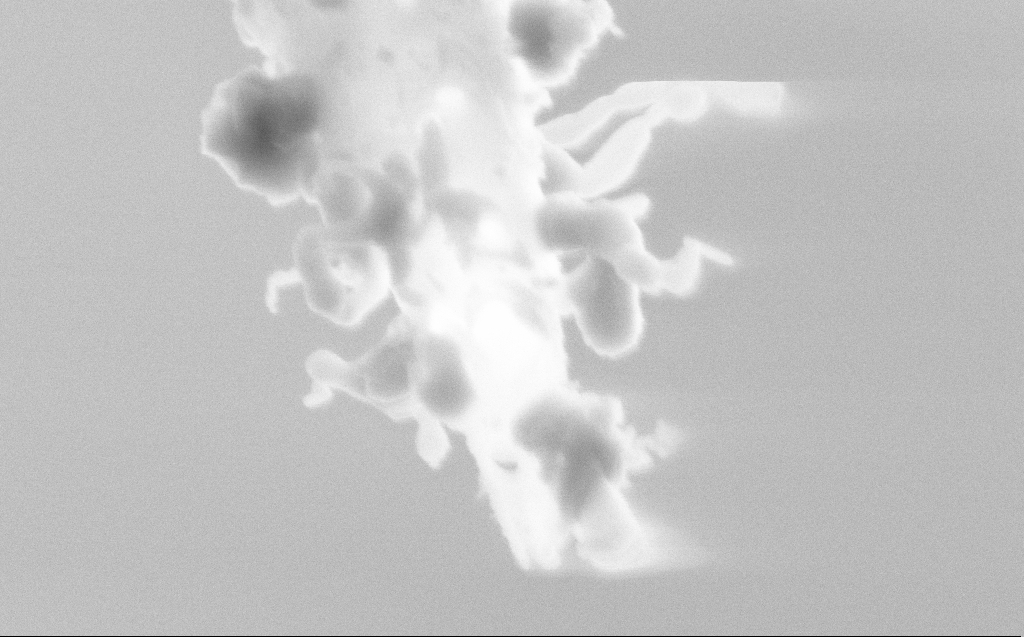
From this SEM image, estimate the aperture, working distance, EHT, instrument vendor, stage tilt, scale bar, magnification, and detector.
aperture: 30 µm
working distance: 4 mm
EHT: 5 kV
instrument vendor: Zeiss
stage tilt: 45°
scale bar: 200 nm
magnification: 178.66 K X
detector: SE2